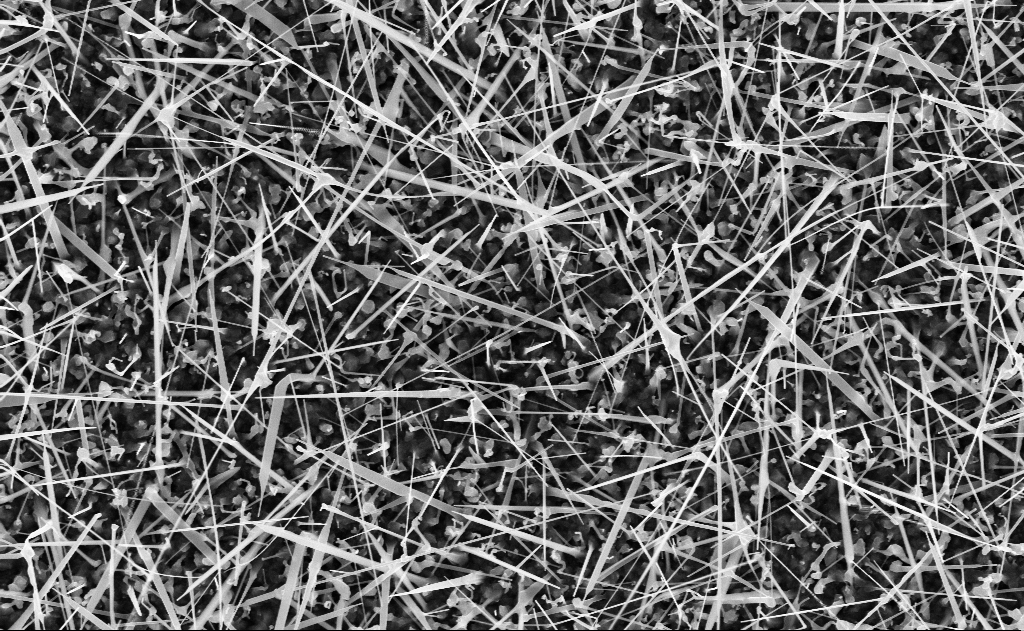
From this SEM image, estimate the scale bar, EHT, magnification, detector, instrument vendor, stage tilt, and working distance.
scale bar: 1000 nm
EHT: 10 kV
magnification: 20 K X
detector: InLens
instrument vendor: Zeiss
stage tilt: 0°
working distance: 11 mm